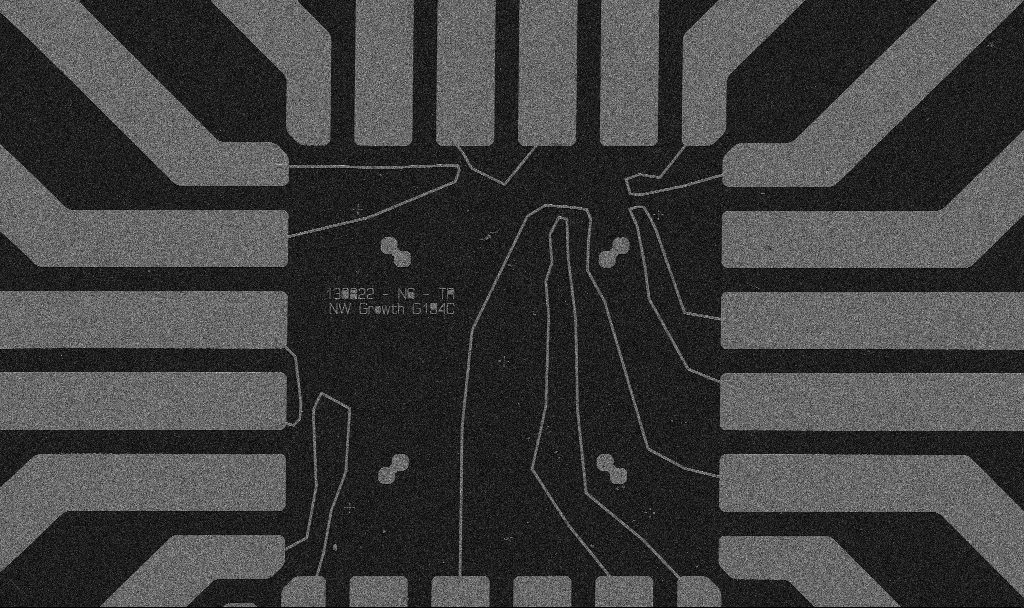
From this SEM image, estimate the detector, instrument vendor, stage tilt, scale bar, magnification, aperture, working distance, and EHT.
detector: SE2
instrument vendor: Zeiss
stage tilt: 0°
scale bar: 20000 nm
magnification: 1 K X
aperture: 30 µm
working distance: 10.7 mm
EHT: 5 kV